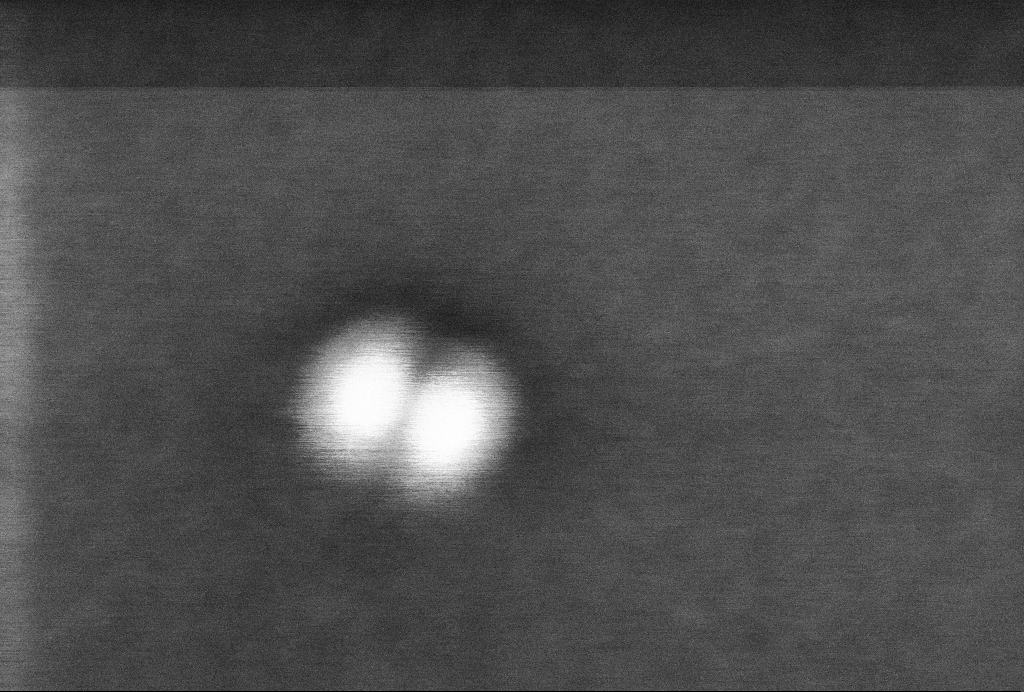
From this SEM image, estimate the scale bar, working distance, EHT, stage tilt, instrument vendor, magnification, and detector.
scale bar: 20 nm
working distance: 3.3 mm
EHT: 2 kV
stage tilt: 0°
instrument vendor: Zeiss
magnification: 1356.85 K X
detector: InLens